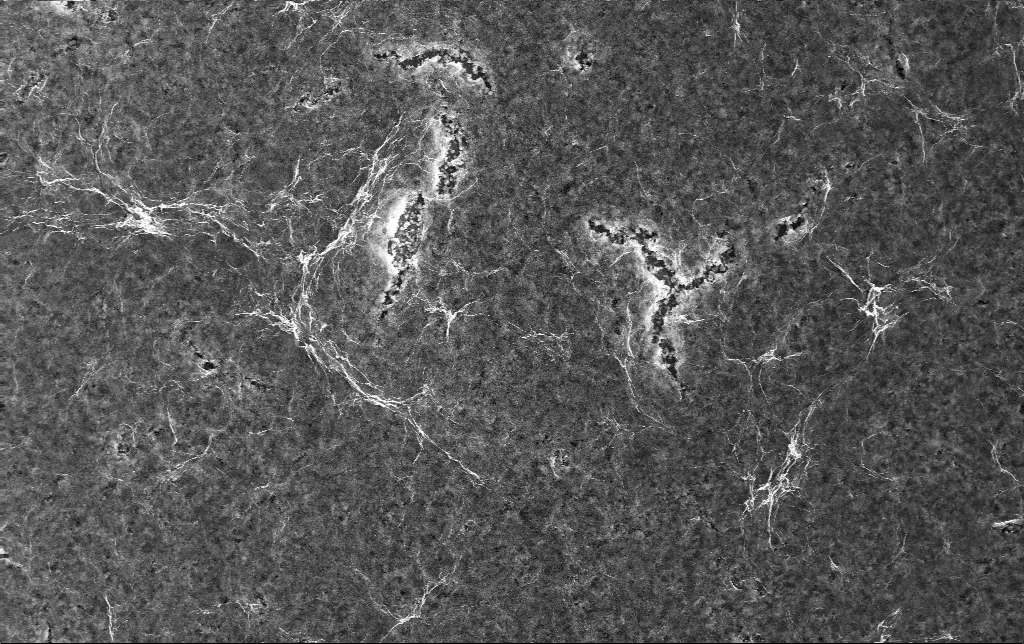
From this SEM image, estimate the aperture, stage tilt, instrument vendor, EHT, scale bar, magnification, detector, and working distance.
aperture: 30 µm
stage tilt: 0°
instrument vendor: Zeiss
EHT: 10 kV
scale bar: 10000 nm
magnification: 5 K X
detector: InLens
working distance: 3 mm